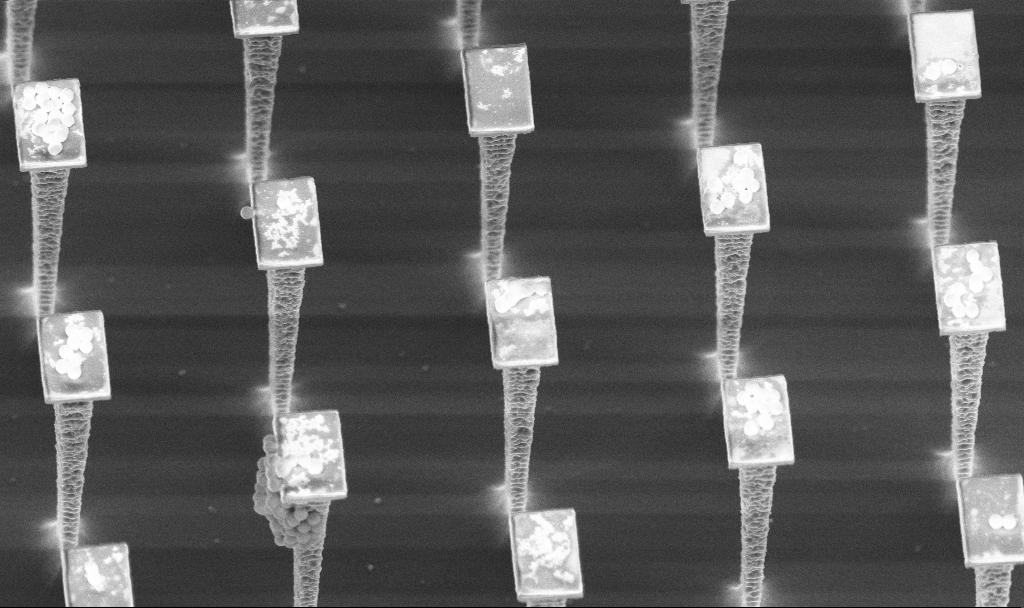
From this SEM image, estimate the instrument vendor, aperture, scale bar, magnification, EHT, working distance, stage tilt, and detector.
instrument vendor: Zeiss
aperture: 30 µm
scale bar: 2000 nm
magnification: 8.21 K X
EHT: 5 kV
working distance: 4.5 mm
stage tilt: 30°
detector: InLens